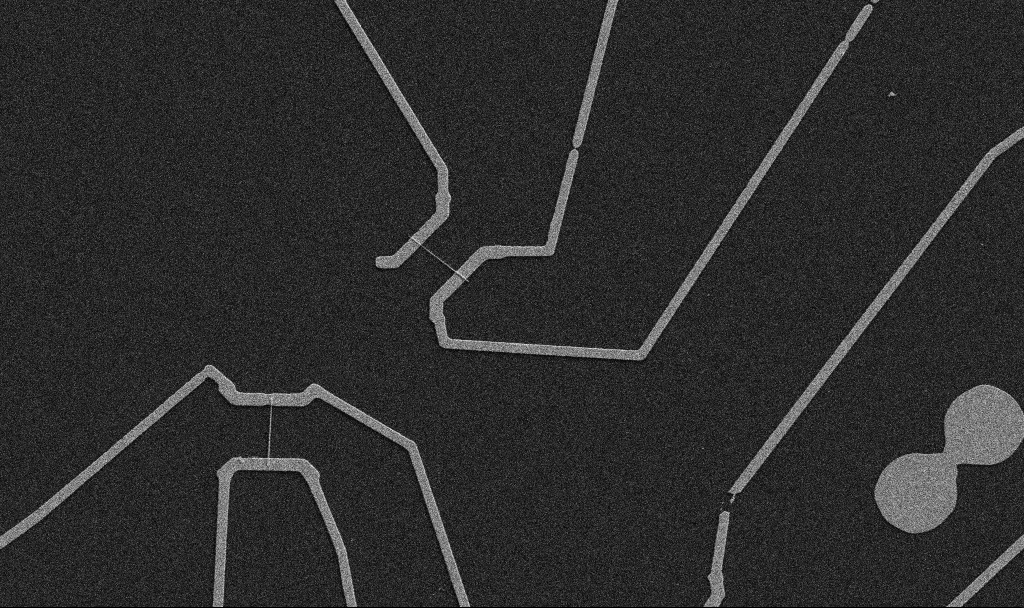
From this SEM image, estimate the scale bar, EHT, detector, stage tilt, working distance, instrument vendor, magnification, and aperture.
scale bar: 10000 nm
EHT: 5 kV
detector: SE2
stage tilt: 0°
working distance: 10.7 mm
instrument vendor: Zeiss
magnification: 5 K X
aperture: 30 µm